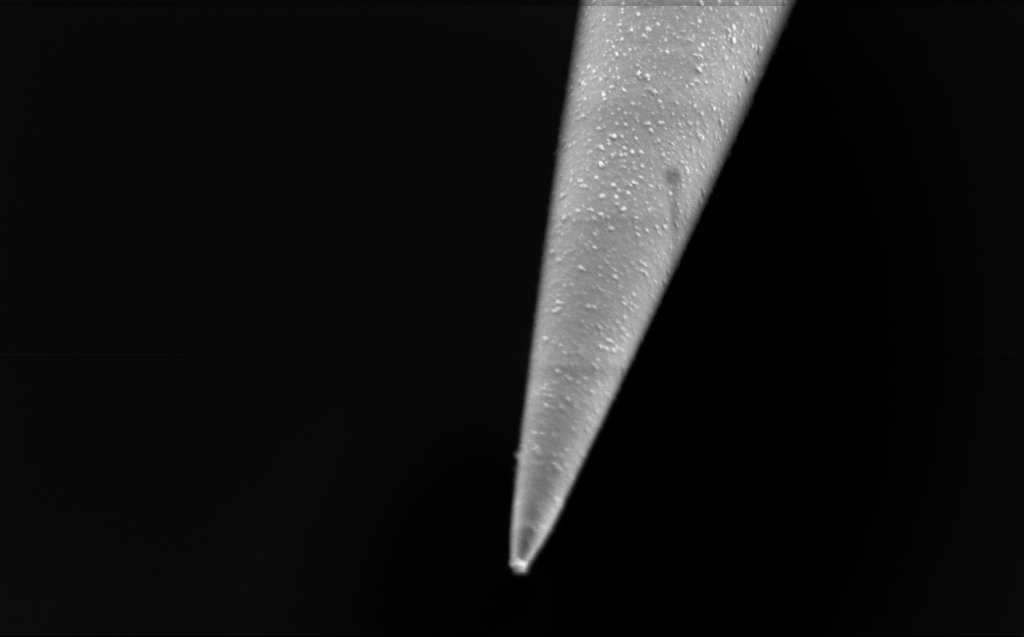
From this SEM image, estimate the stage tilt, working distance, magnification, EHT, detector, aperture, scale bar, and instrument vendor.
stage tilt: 45°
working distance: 3 mm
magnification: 54.89 K X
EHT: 1 kV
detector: InLens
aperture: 30 µm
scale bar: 1000 nm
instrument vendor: Zeiss